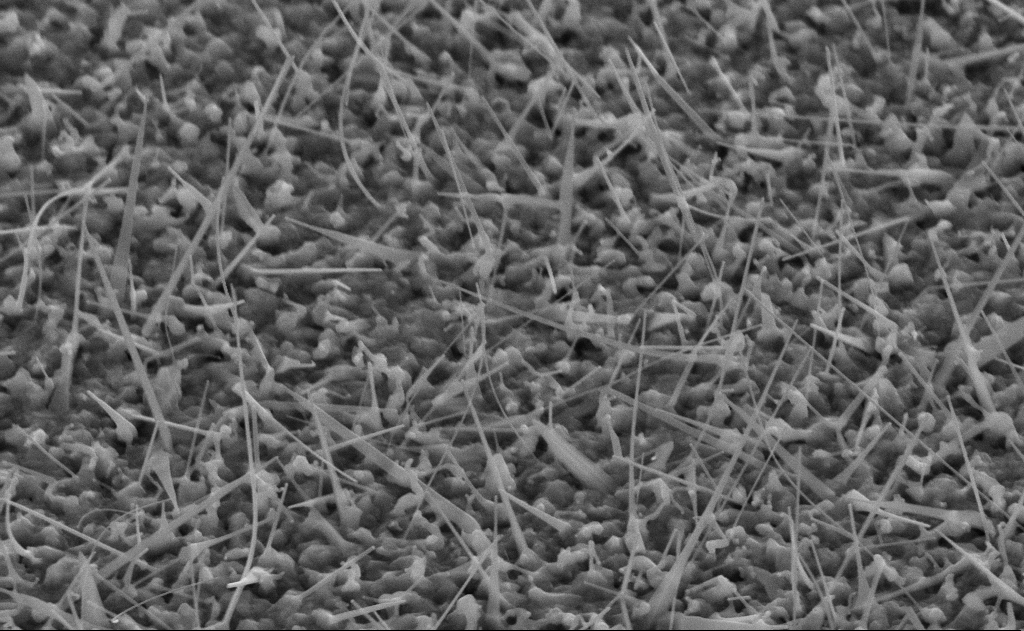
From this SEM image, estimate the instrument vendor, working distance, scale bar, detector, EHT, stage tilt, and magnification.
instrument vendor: Zeiss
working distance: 11 mm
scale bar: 1000 nm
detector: SE2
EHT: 10 kV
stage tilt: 45°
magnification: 40 K X